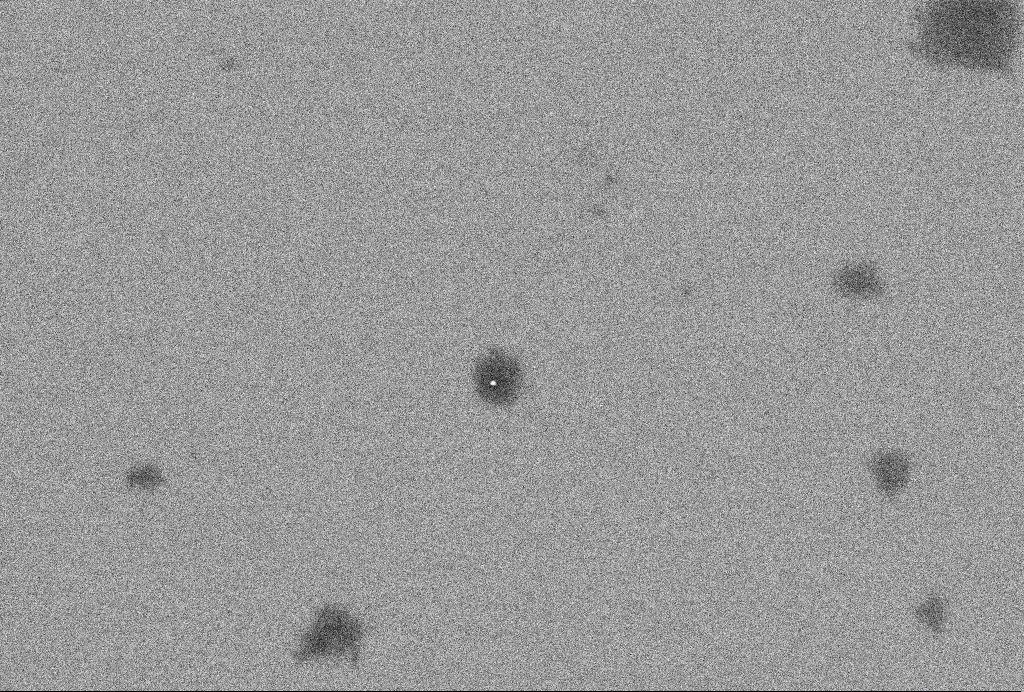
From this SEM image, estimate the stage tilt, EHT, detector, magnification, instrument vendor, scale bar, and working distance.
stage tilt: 0°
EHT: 2 kV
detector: InLens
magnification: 64.42 K X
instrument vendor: Zeiss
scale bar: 200 nm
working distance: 3.3 mm